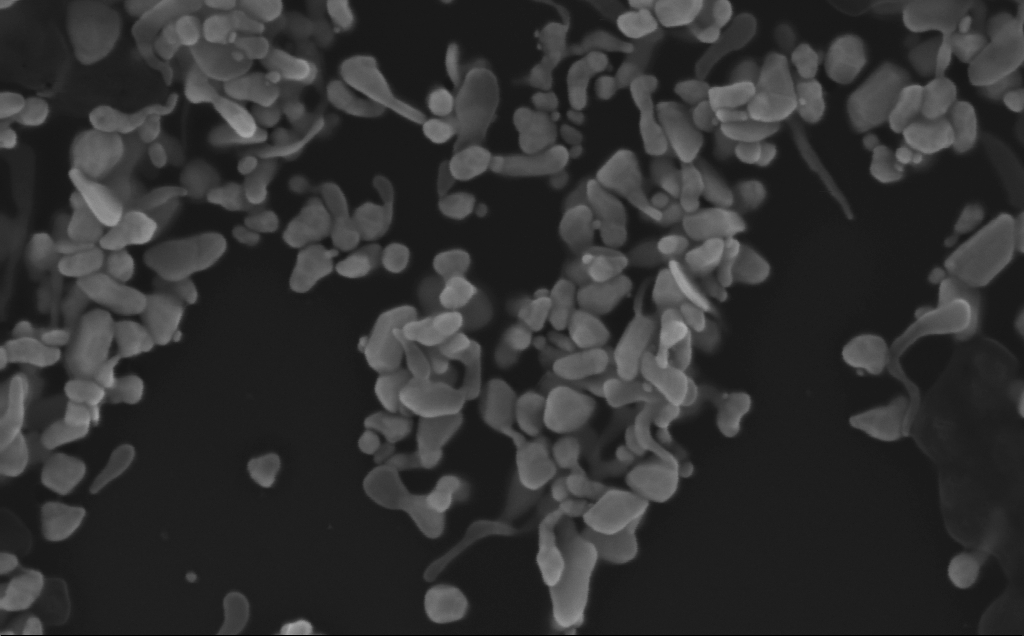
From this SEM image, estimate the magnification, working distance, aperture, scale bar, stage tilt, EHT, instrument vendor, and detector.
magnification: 226.08 K X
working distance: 3 mm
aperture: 30 µm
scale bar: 200 nm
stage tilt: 0°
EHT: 10 kV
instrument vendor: Zeiss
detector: InLens